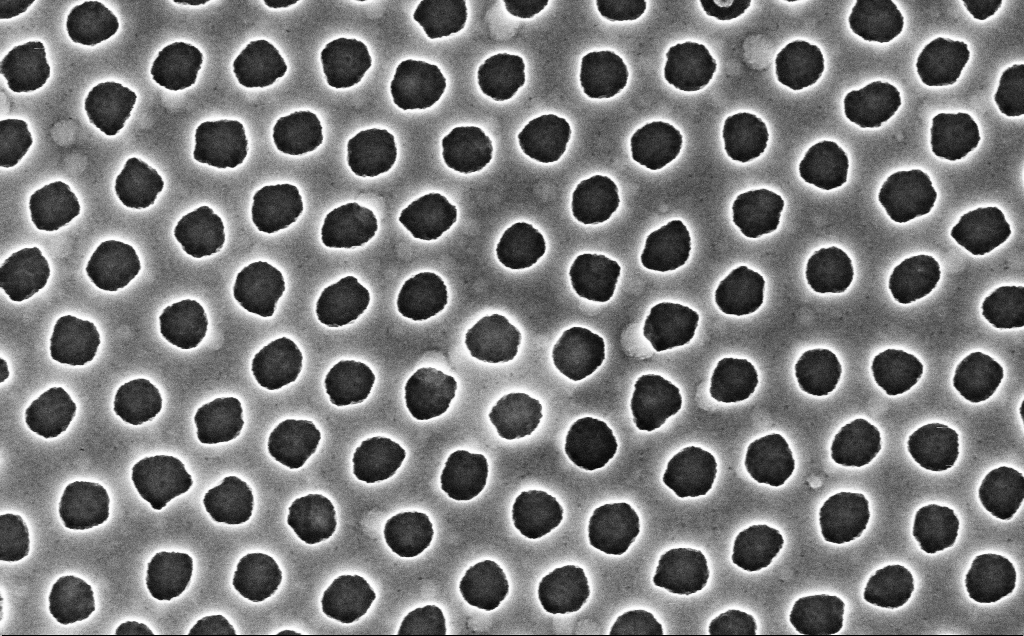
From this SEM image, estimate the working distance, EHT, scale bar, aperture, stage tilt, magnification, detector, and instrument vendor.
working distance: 2.4 mm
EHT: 3 kV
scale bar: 200 nm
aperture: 30 µm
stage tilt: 0°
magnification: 90 K X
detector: InLens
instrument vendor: Zeiss